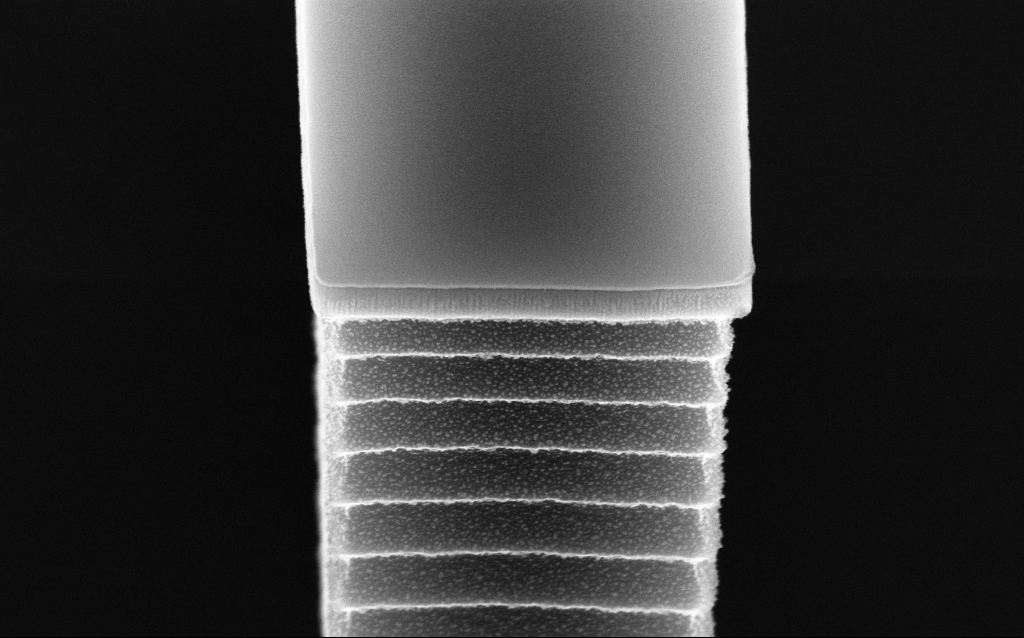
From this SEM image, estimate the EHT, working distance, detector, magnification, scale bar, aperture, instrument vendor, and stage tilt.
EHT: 10 kV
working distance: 4.8 mm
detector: InLens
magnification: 80.77 K X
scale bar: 200 nm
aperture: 30 µm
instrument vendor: Zeiss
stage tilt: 45°